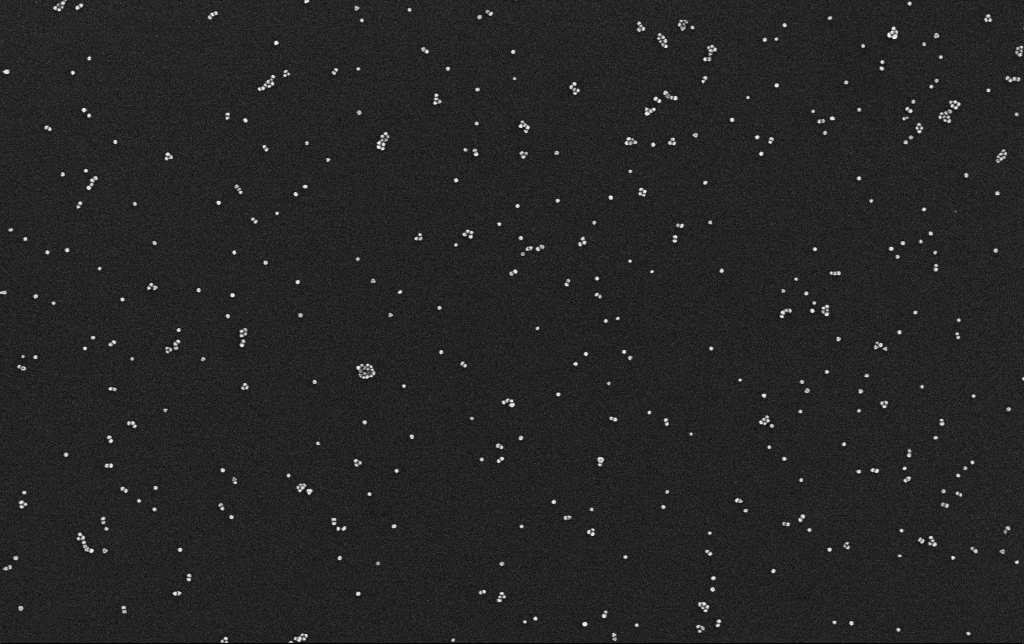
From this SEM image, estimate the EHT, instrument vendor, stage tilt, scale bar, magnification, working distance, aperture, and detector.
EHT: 10 kV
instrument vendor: Zeiss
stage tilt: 0°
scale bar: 200 nm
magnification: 100 K X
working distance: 3.1 mm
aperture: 30 µm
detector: InLens